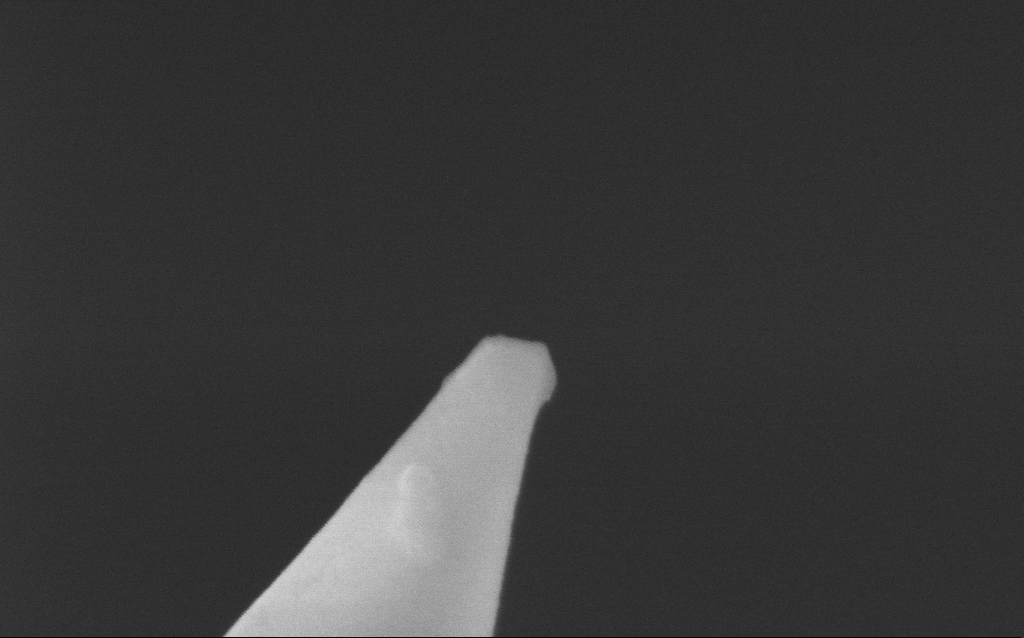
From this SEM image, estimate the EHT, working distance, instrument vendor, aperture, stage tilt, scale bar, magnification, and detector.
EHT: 5 kV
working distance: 4.9 mm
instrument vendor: Zeiss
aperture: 30 µm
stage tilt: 0°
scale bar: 100 nm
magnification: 250 K X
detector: InLens